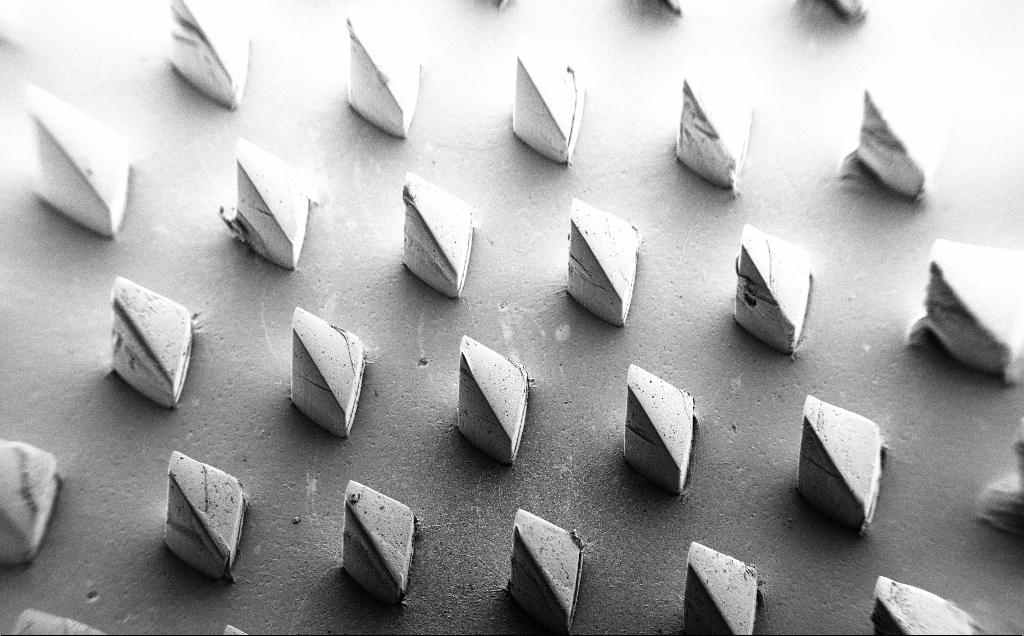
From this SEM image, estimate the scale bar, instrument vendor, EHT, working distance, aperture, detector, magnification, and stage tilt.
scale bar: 1e+06 nm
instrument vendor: Zeiss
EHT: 5 kV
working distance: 8 mm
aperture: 30 µm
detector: SE2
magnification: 0.067 K X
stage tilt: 39°